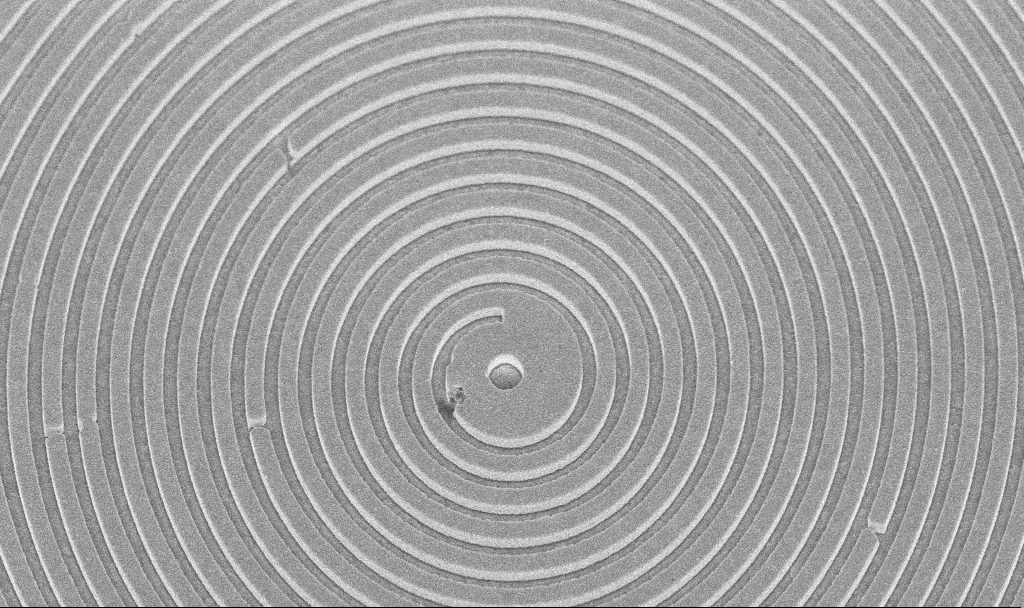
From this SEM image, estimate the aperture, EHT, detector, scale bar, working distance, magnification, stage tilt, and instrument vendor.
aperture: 30 µm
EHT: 5 kV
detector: InLens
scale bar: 2000 nm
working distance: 8.4 mm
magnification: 19.21 K X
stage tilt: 45°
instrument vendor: Zeiss